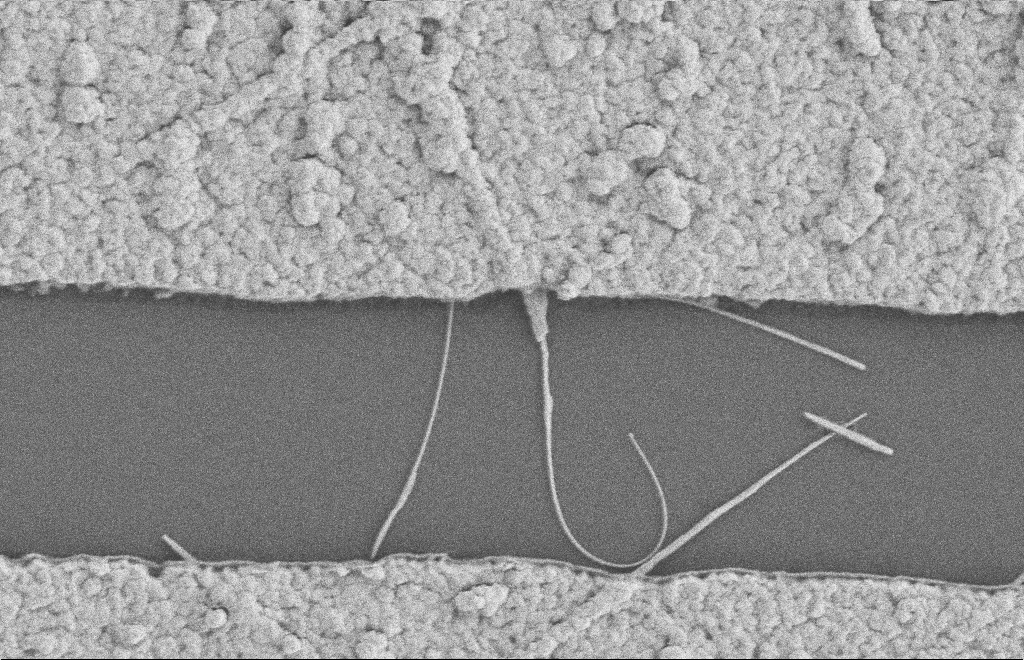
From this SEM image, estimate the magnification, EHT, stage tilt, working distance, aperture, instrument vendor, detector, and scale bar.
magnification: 38.43 K X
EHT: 2 kV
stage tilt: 0°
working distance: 8 mm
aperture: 20 µm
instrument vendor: Zeiss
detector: SE2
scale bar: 1000 nm